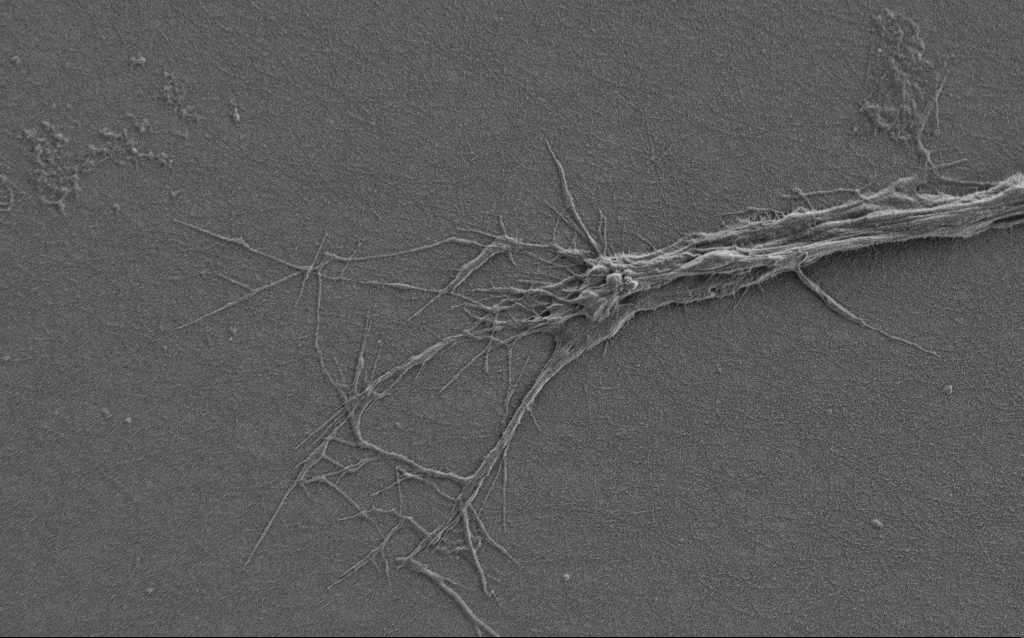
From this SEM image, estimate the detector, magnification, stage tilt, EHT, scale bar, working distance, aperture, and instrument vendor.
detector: SE2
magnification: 6 K X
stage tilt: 0°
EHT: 0.9 kV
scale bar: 10000 nm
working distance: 6 mm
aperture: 30 µm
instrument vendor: Zeiss